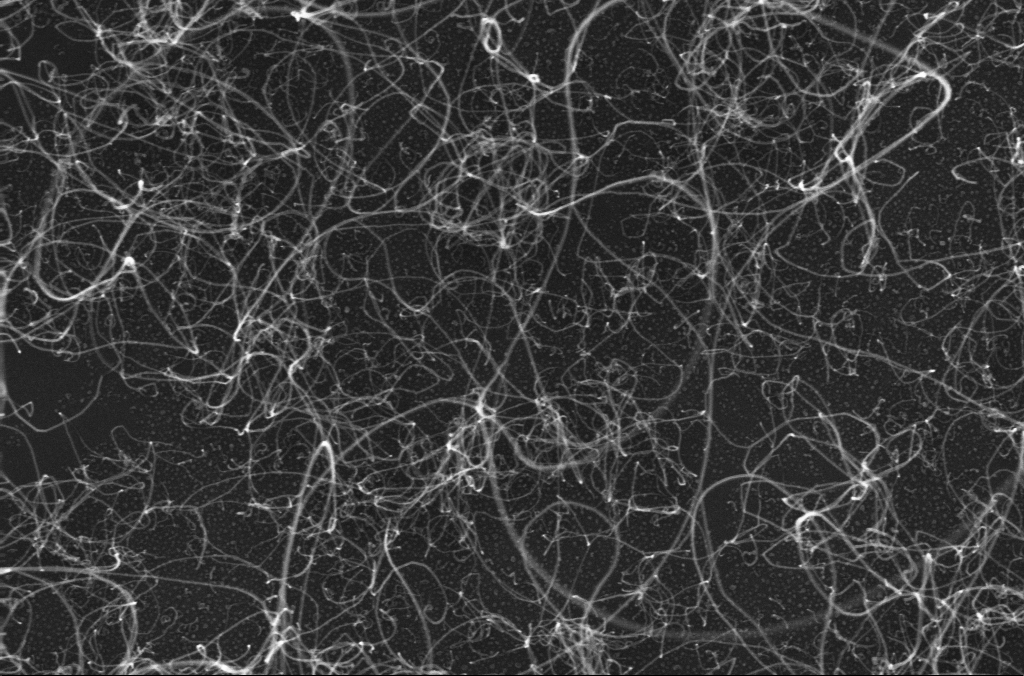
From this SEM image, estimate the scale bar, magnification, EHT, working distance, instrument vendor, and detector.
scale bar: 200 nm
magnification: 100 K X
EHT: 10 kV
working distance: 3.2 mm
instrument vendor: Zeiss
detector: InLens